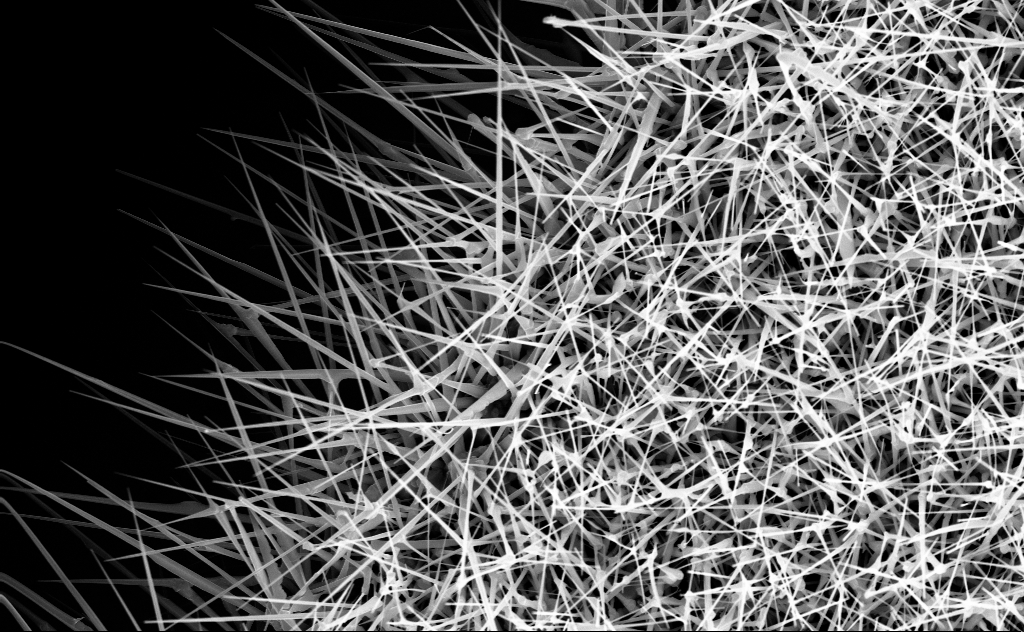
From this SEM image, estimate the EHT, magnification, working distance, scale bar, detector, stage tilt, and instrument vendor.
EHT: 10 kV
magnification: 14.31 K X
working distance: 6 mm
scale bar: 2000 nm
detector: InLens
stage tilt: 0°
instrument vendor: Zeiss